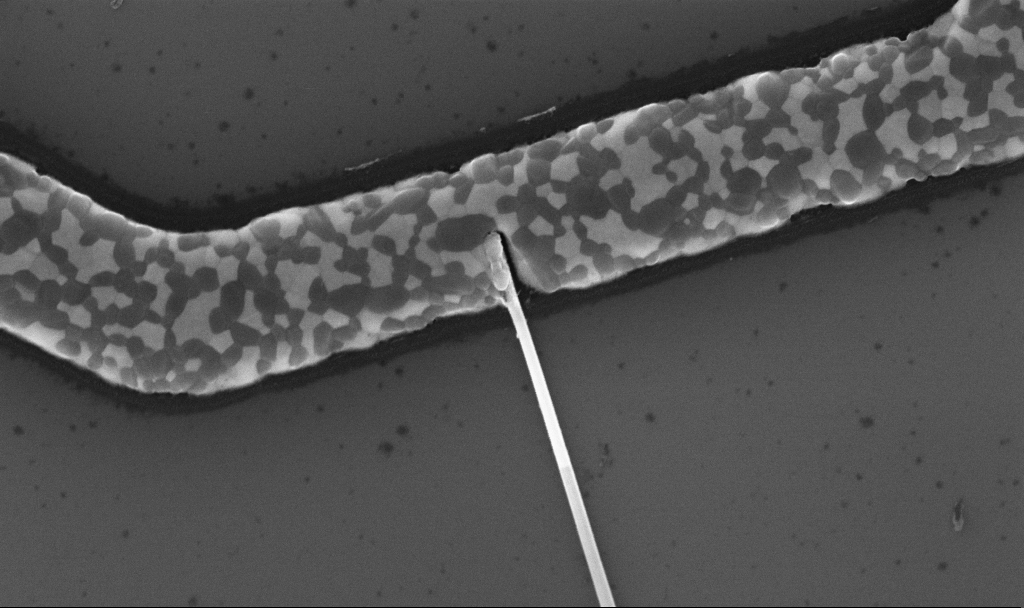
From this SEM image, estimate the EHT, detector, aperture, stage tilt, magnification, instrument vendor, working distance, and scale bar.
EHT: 10 kV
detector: InLens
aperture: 30 µm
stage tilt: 0°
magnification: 64.72 K X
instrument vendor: Zeiss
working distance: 6.7 mm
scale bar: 1000 nm